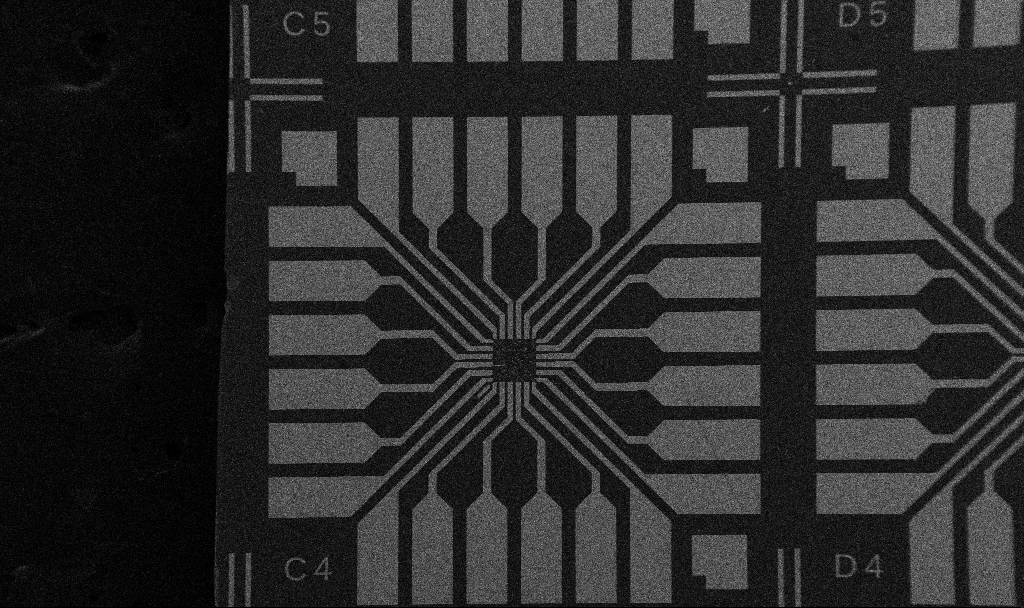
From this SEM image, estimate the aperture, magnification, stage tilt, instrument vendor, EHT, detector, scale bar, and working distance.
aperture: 30 µm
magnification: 0.1 K X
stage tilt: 0°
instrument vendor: Zeiss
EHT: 5 kV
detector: SE2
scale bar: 200000 nm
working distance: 10.7 mm